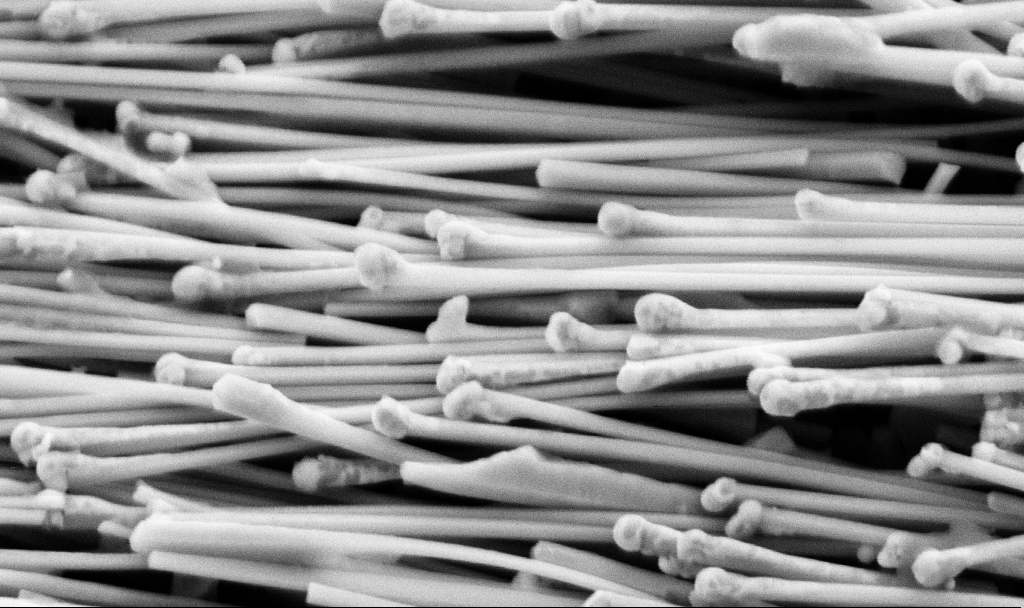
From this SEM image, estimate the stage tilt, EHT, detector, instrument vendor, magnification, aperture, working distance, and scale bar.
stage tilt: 45°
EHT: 5 kV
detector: SE2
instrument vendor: Zeiss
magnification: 60.68 K X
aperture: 30 µm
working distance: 11.2 mm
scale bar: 1000 nm